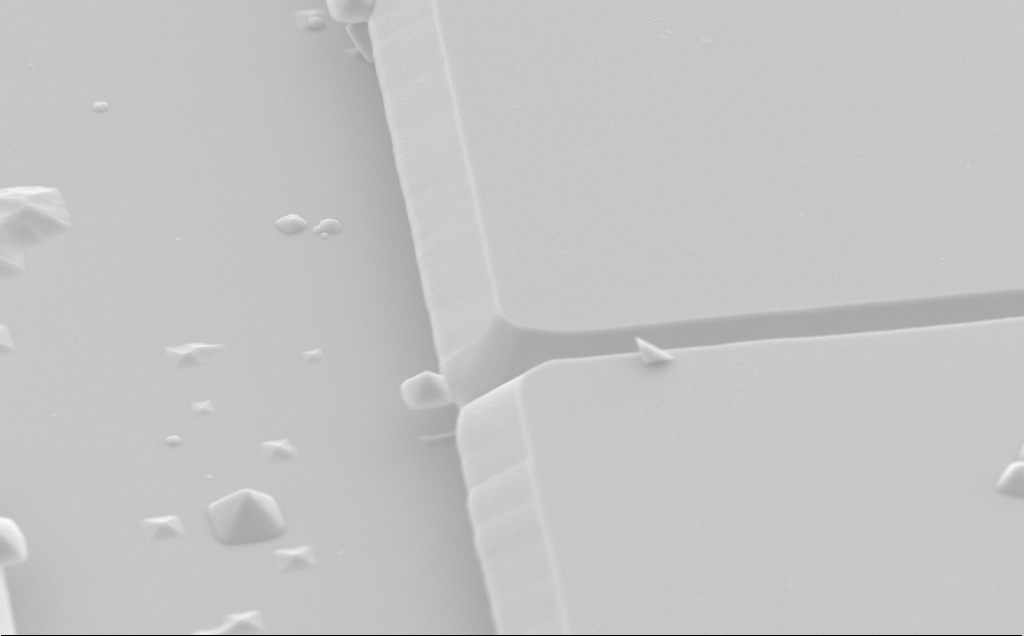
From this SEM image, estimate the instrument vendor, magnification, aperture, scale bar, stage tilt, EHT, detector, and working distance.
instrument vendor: Zeiss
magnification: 11.11 K X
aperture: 30 µm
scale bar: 2000 nm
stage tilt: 50°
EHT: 5 kV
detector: SE2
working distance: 10 mm